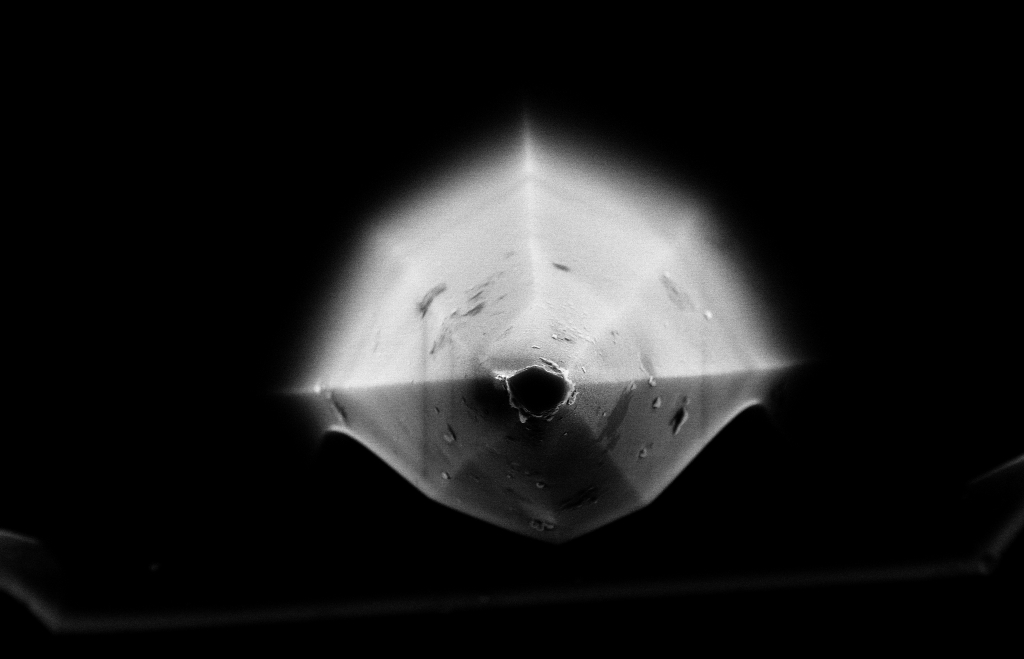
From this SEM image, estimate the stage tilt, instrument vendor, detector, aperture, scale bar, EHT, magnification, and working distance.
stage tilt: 0°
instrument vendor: Zeiss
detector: InLens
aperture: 30 µm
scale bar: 1000 nm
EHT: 10 kV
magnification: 15.6 K X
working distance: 8 mm